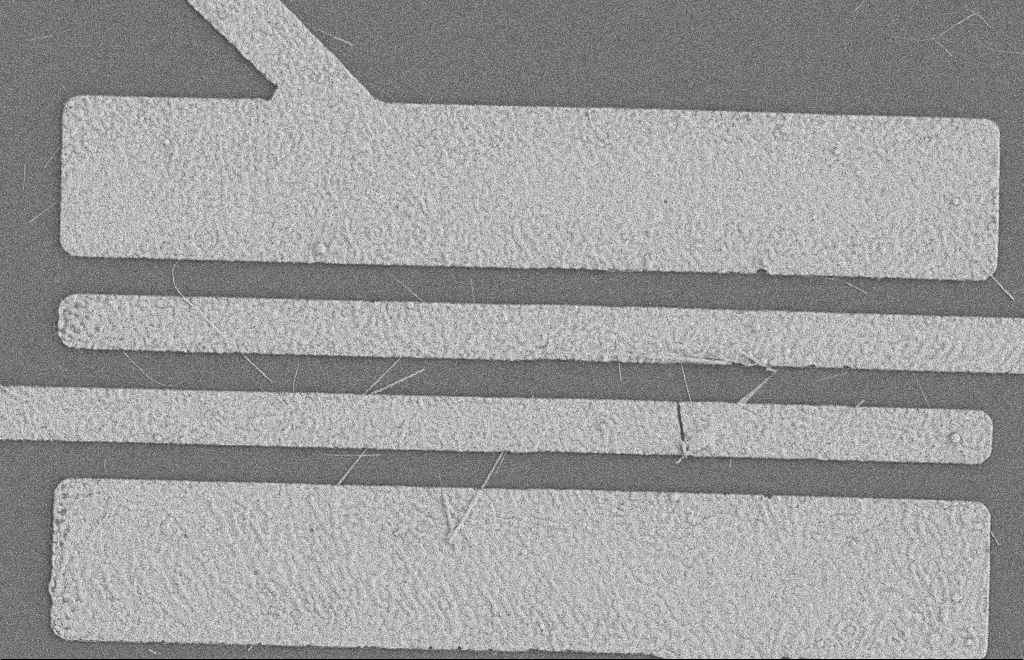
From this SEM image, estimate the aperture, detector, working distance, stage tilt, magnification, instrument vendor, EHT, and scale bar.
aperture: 20 µm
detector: SE2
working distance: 8 mm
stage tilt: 0°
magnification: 5.63 K X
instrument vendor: Zeiss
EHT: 2 kV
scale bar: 2000 nm